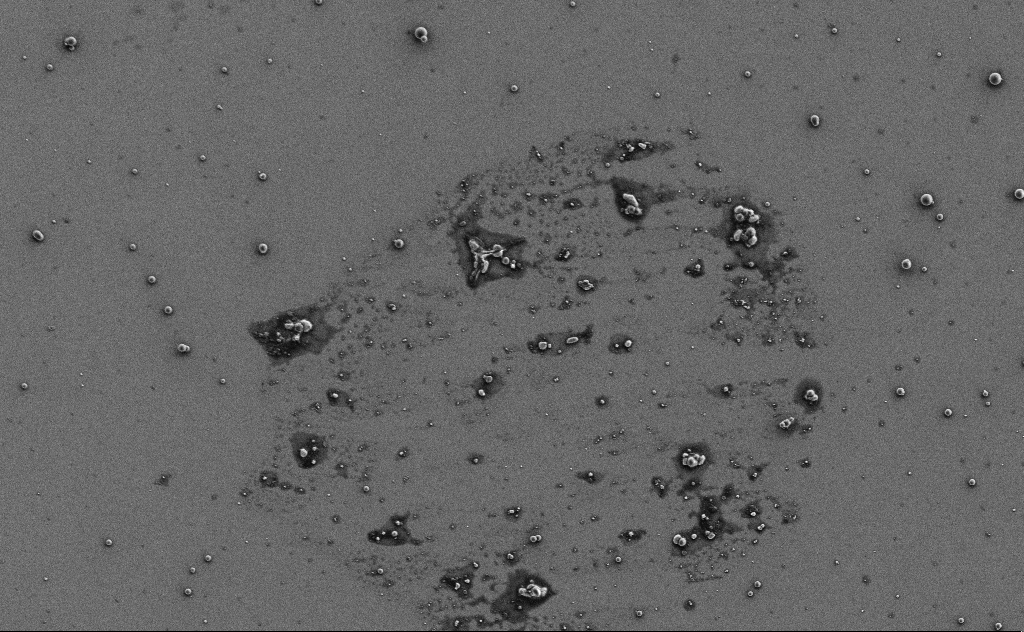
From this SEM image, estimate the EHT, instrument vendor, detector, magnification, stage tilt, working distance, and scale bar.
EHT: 3 kV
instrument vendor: Zeiss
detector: SE2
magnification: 2.34 K X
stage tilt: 0°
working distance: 11 mm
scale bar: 20000 nm